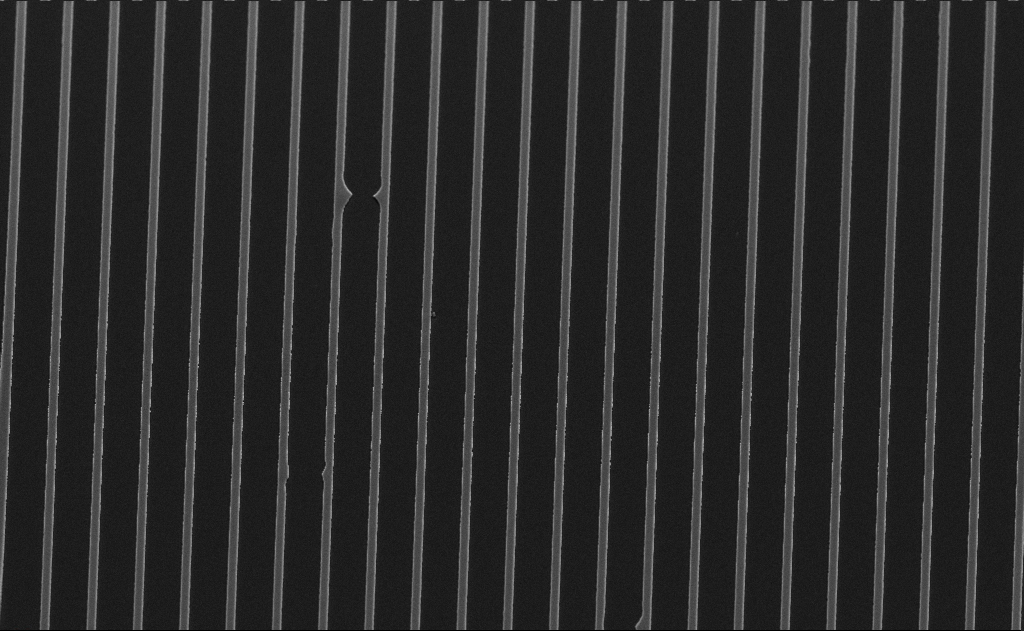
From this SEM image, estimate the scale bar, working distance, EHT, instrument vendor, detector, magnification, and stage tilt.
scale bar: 20000 nm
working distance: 12 mm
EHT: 6 kV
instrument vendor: Zeiss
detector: SE2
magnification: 2.12 K X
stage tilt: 50°